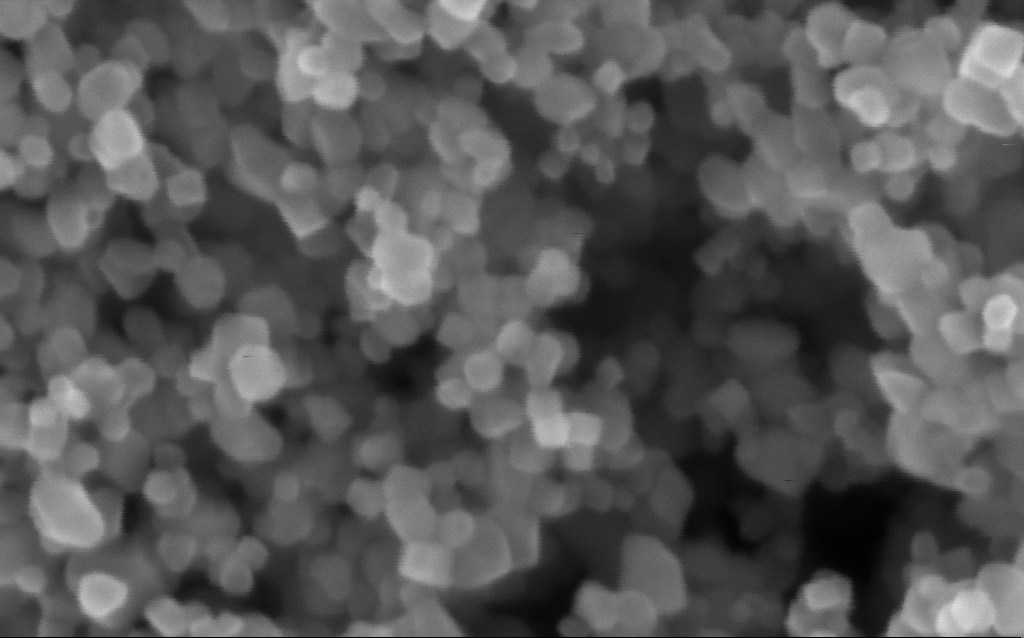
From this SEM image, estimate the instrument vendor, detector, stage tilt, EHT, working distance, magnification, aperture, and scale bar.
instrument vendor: Zeiss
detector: InLens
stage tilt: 0°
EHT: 5 kV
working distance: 2.9 mm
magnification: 716 K X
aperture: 30 µm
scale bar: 100 nm